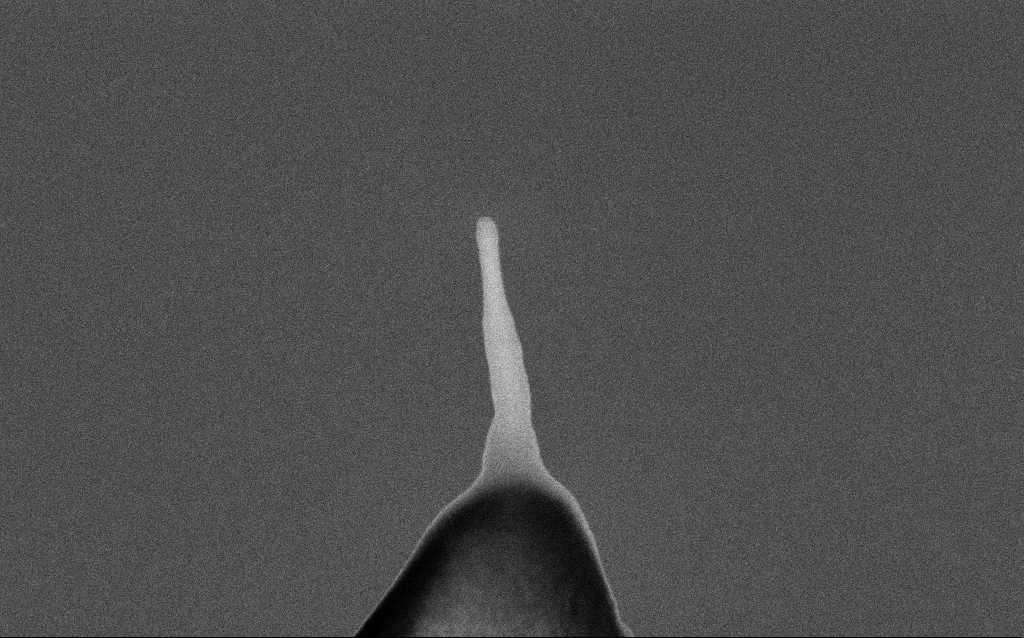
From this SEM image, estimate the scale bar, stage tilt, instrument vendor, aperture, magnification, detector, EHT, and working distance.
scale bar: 100 nm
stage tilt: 0°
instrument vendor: Zeiss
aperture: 30 µm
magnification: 150.04 K X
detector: InLens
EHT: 5 kV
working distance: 3 mm